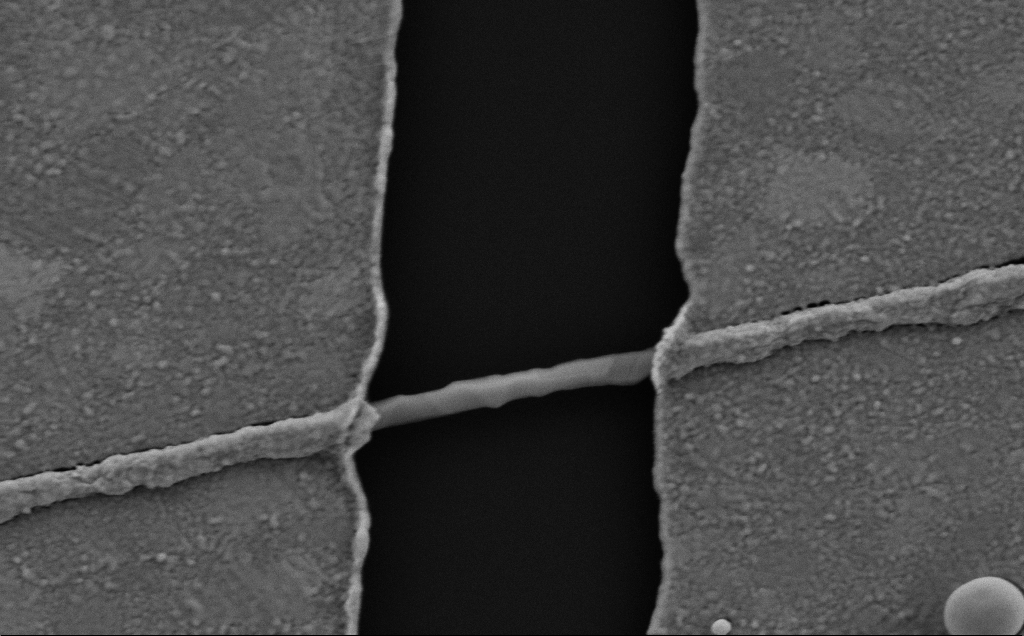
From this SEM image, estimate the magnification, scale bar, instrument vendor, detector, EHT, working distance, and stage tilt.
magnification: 73.37 K X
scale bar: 200 nm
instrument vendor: Zeiss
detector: SE2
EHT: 5 kV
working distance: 6 mm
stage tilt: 0°